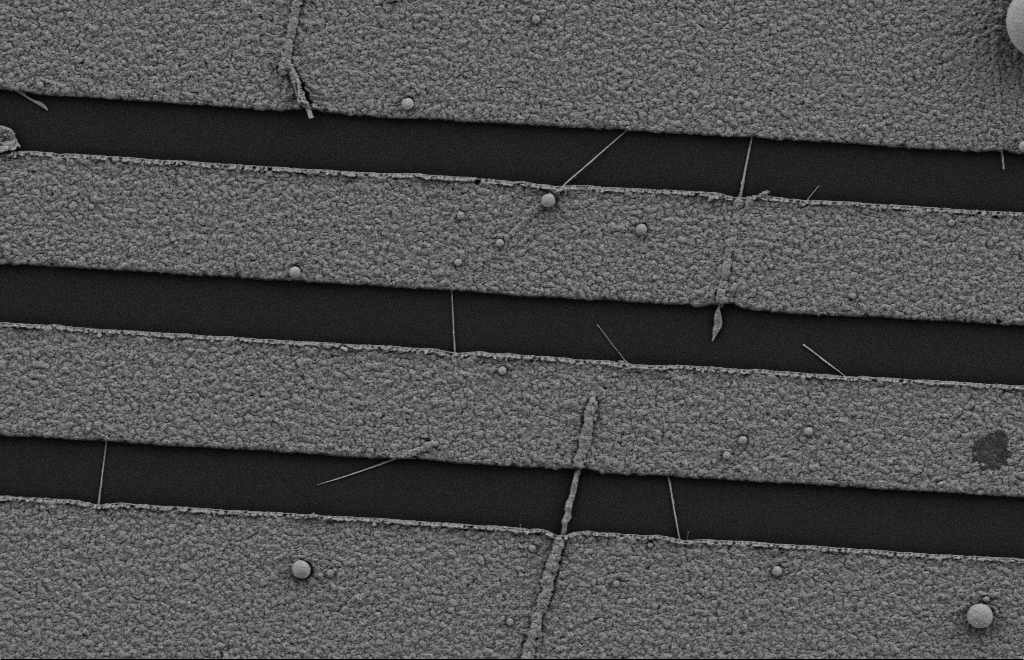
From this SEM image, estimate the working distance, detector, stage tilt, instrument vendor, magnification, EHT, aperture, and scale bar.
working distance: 10 mm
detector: SE2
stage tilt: -0.3°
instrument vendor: Zeiss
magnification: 15.75 K X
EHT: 2 kV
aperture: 20 µm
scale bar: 2000 nm